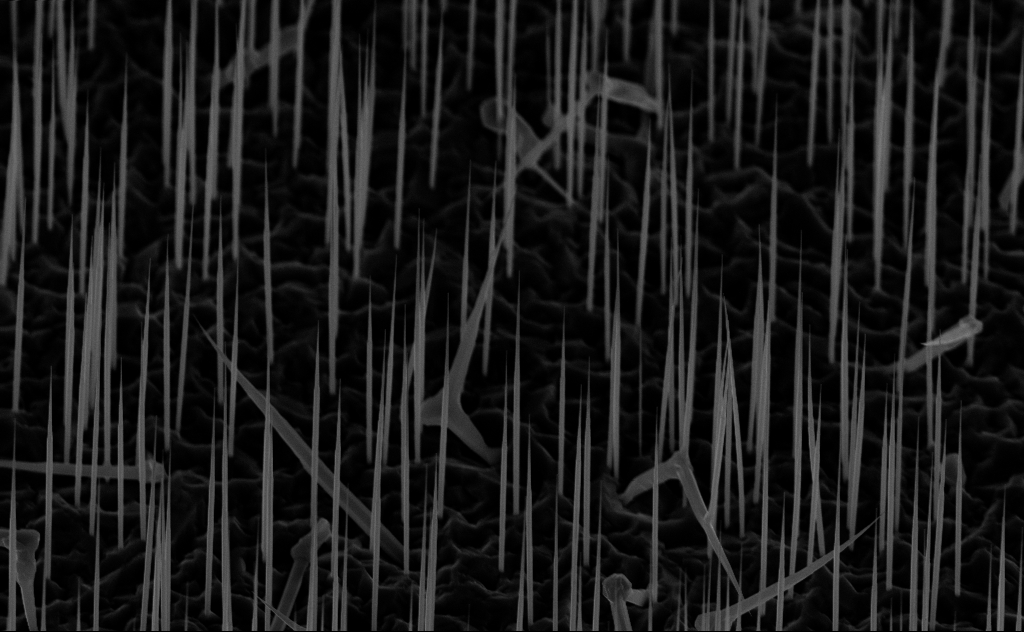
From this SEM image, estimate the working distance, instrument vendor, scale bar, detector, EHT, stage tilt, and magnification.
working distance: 7 mm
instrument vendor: Zeiss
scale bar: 2000 nm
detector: InLens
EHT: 10 kV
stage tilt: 45°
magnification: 14.49 K X